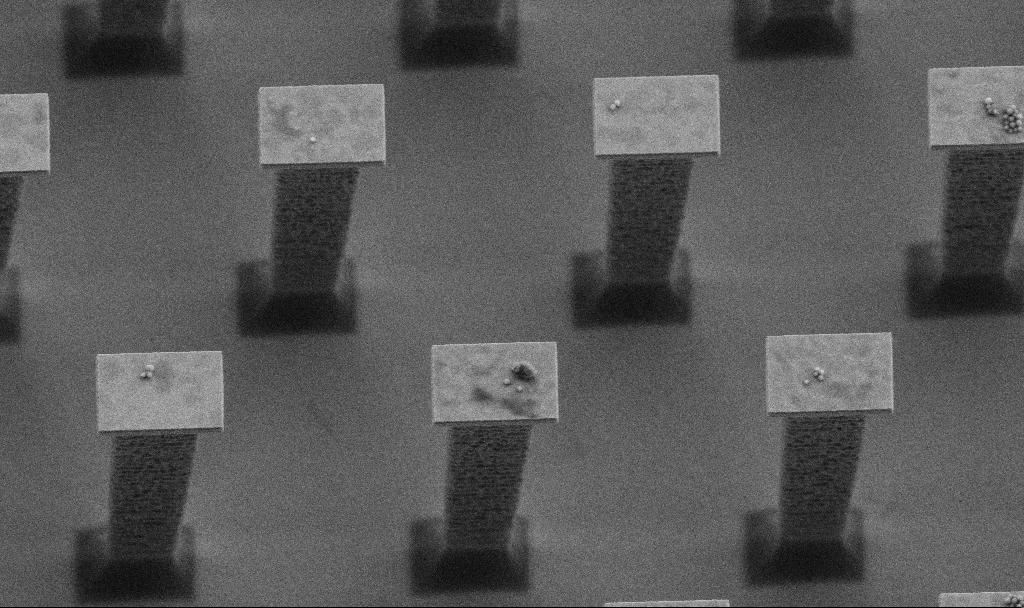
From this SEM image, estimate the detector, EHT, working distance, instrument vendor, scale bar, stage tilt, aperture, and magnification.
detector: SE2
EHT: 3 kV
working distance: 5.6 mm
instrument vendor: Zeiss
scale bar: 2000 nm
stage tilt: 20°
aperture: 30 µm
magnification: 10.19 K X